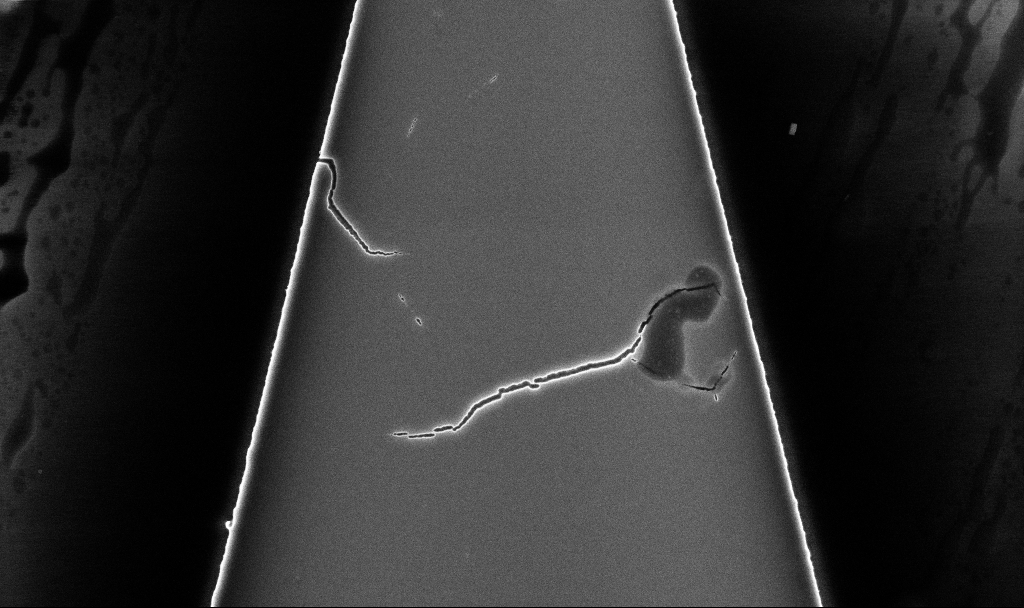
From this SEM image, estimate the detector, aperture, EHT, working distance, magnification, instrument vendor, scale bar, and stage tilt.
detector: InLens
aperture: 30 µm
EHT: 5 kV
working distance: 5.2 mm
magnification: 15.72 K X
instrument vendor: Zeiss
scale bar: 2000 nm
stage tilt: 0°